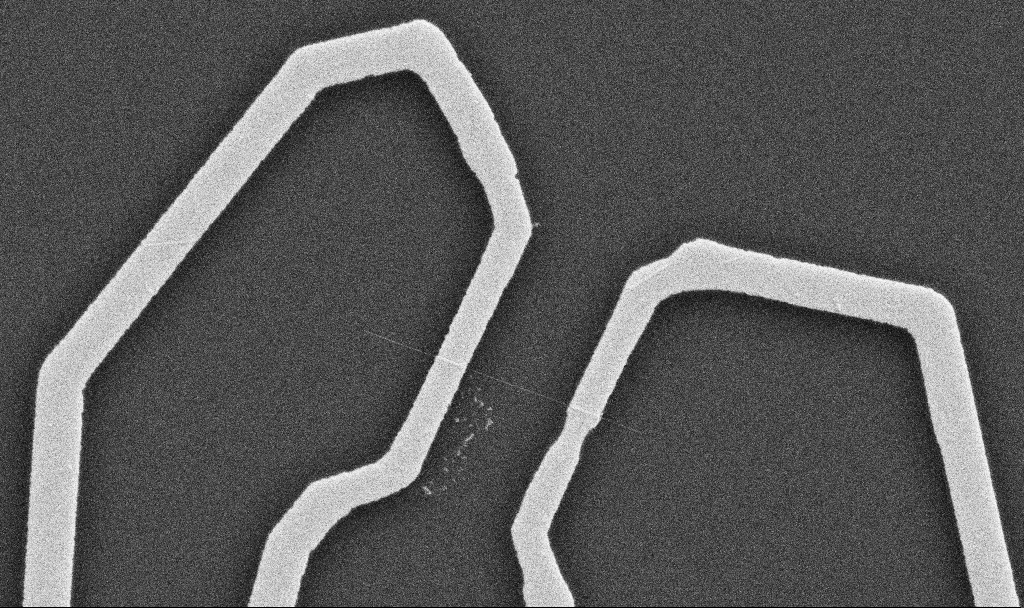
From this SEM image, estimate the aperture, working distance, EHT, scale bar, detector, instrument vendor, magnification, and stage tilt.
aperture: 30 µm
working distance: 10.7 mm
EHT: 10 kV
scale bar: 1000 nm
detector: SE2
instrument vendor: Zeiss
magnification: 20 K X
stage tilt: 0°